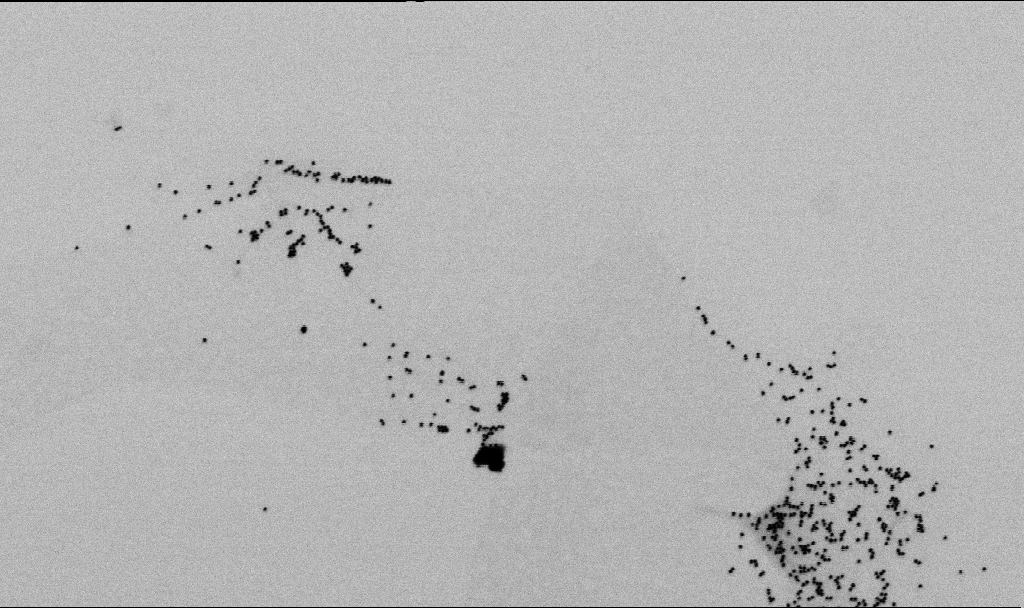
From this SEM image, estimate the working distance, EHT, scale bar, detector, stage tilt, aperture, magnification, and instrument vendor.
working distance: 6.5 mm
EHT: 2 kV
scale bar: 1000 nm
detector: SE2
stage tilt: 0°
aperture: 30 µm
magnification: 60 K X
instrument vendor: Zeiss